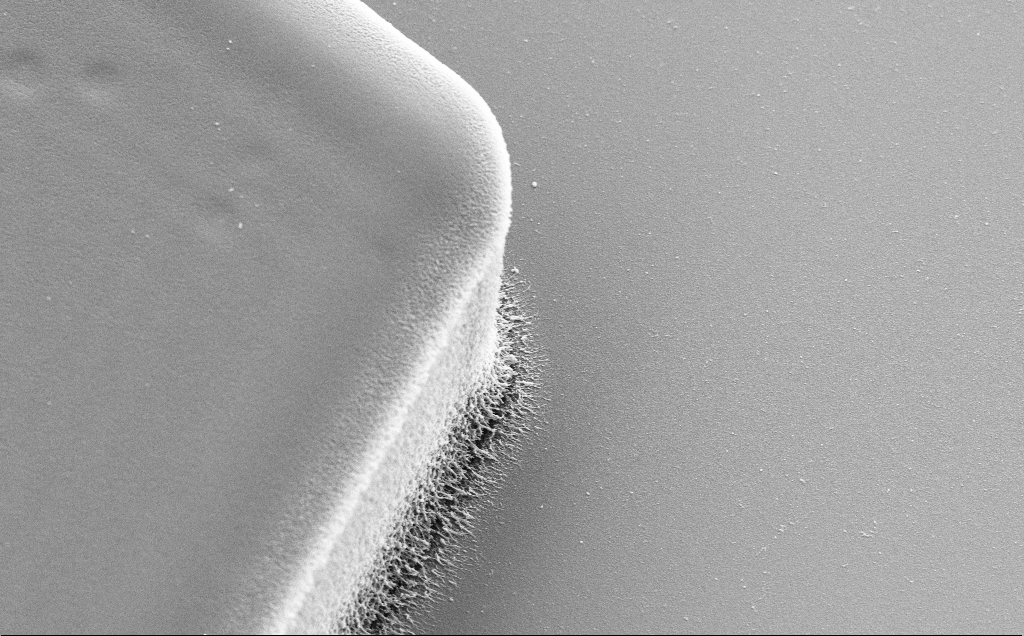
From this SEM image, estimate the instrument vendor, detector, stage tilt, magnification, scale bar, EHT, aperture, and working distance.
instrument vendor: Zeiss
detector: SE2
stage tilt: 30°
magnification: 14.13 K X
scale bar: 1000 nm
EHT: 5 kV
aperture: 30 µm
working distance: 10 mm